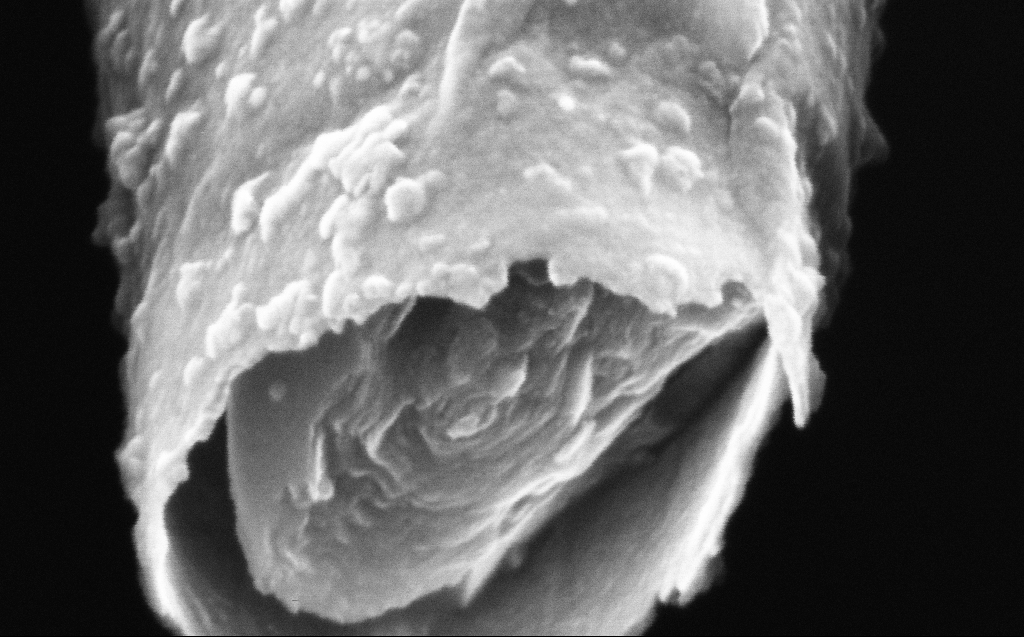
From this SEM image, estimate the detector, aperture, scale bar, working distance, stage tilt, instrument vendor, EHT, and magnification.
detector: InLens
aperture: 30 µm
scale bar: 100 nm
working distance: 4 mm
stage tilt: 45°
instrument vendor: Zeiss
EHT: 4 kV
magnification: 400 K X